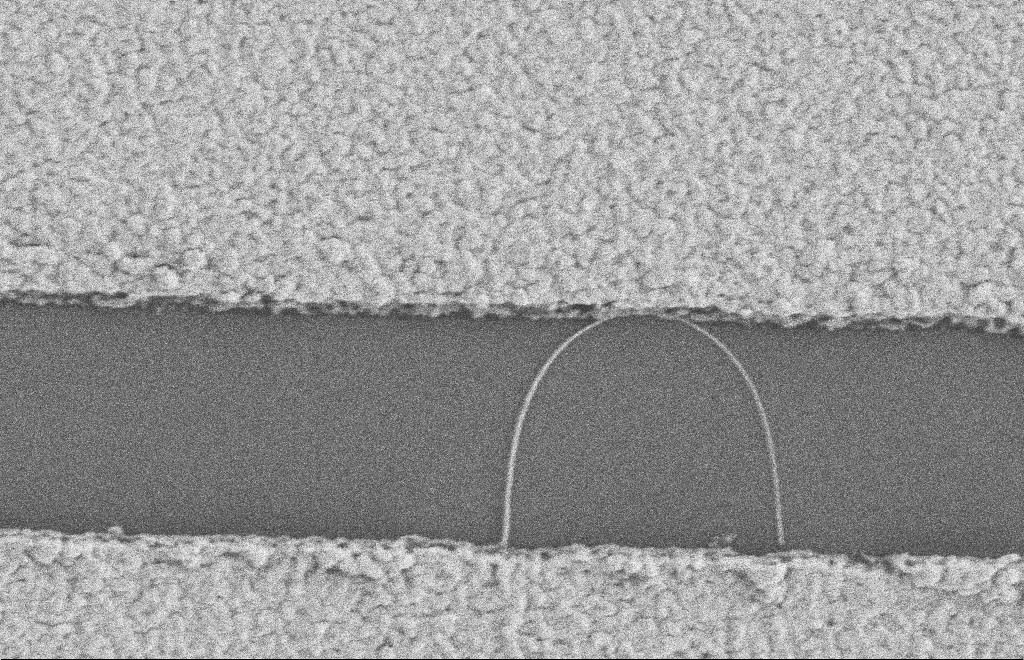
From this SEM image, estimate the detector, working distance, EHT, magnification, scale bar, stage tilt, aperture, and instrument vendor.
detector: SE2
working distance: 8 mm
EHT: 2 kV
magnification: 39.38 K X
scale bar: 1000 nm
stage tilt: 0°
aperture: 20 µm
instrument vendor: Zeiss